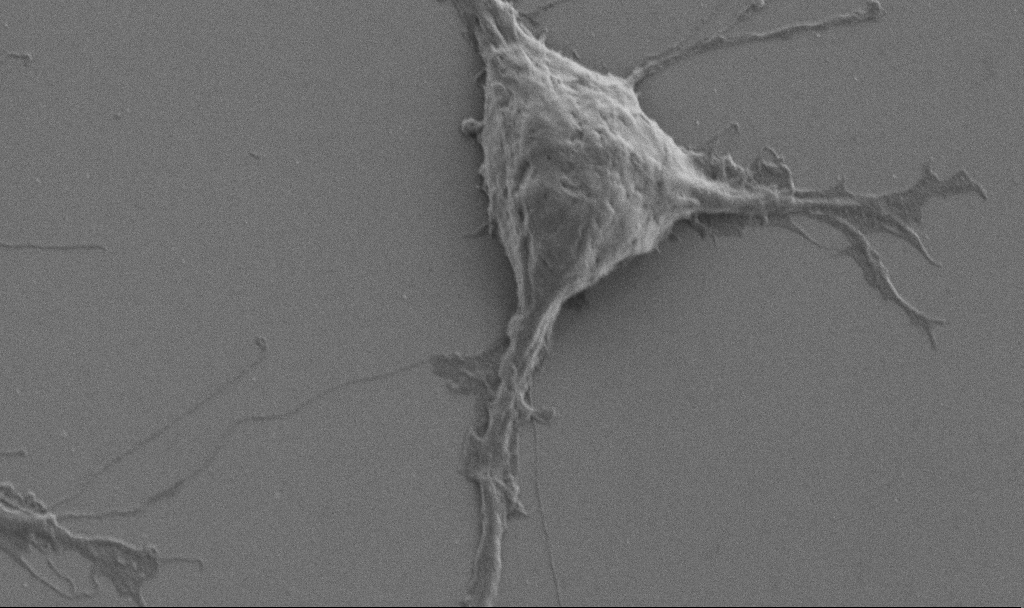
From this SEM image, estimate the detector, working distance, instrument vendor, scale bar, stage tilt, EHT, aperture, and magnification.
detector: SE2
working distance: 6.9 mm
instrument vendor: Zeiss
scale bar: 2000 nm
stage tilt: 0°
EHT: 1 kV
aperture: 30 µm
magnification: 10 K X